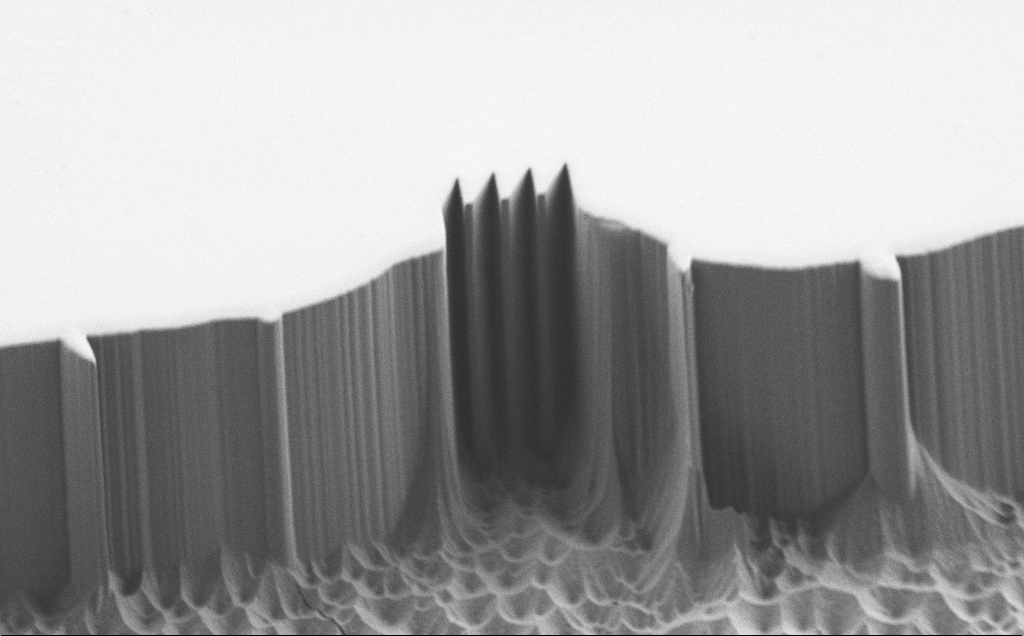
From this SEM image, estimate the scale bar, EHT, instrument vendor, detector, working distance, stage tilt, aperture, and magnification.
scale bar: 20000 nm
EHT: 1.2 kV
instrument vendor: Zeiss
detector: SE2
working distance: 7 mm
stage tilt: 45°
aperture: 30 µm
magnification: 1.13 K X